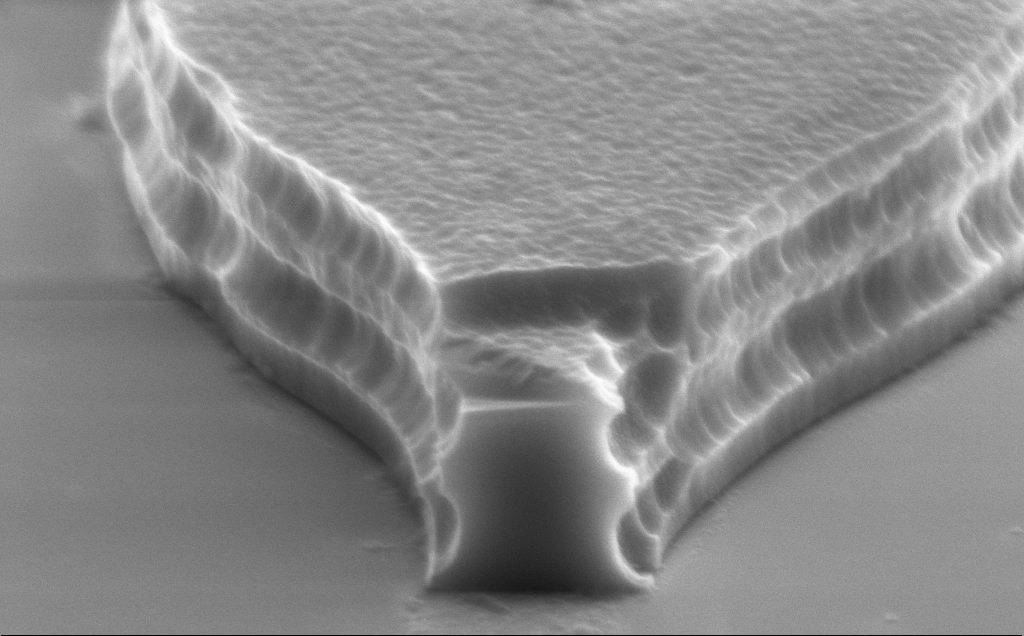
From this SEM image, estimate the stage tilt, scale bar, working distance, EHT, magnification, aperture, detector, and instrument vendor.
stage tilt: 70°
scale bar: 1000 nm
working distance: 12 mm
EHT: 8 kV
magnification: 44.58 K X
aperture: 30 µm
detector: SE2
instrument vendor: Zeiss